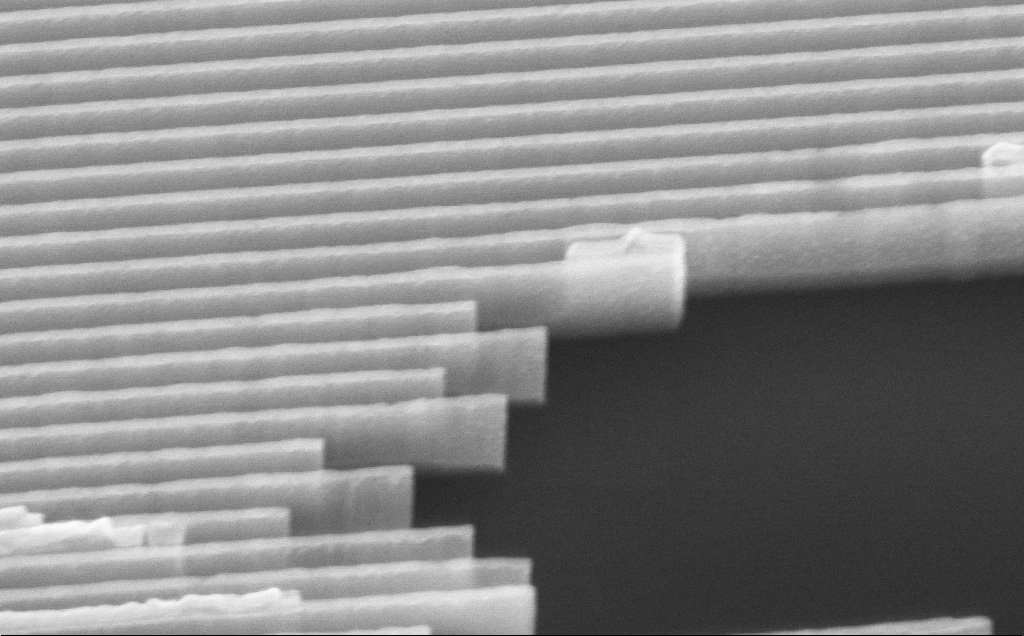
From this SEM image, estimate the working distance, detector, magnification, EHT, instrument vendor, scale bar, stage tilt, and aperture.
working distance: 4 mm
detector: InLens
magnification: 46.55 K X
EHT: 10 kV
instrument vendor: Zeiss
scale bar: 1000 nm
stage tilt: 35°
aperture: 30 µm